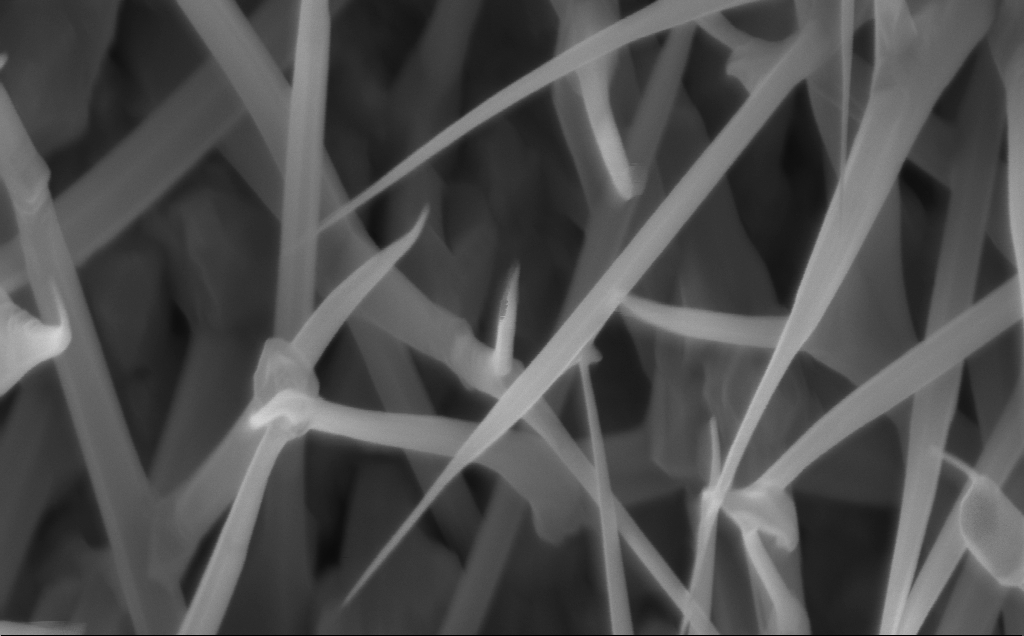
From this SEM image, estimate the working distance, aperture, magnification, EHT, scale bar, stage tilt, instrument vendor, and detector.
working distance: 5 mm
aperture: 30 µm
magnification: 128.62 K X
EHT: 5 kV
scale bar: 200 nm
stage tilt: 0°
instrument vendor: Zeiss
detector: InLens